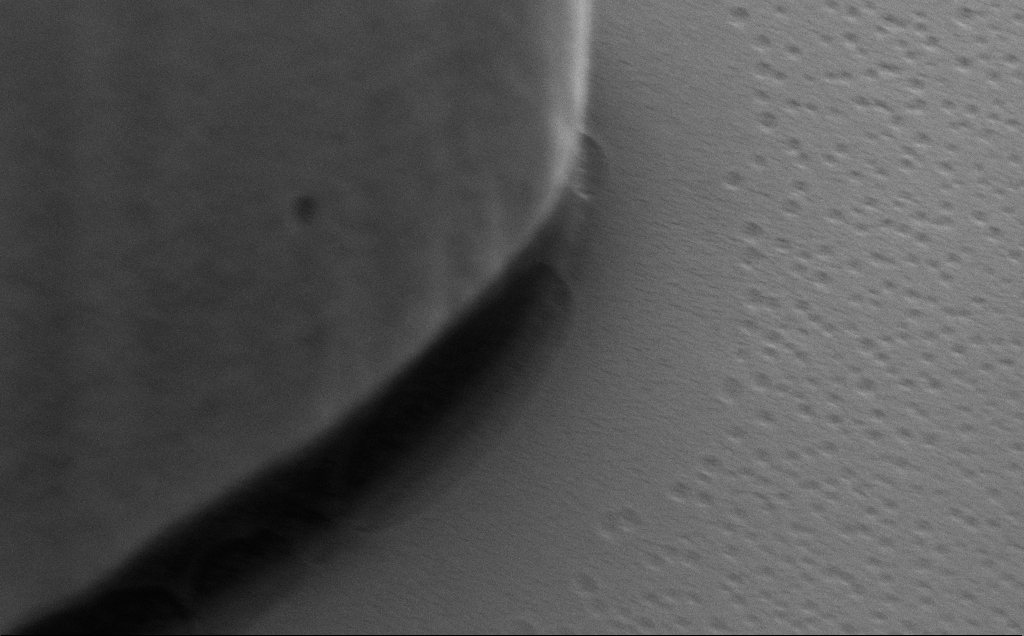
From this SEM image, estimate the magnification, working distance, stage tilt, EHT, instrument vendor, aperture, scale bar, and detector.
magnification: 50 K X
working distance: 8 mm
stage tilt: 40°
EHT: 5 kV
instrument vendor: Zeiss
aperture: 30 µm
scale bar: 1000 nm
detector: SE2